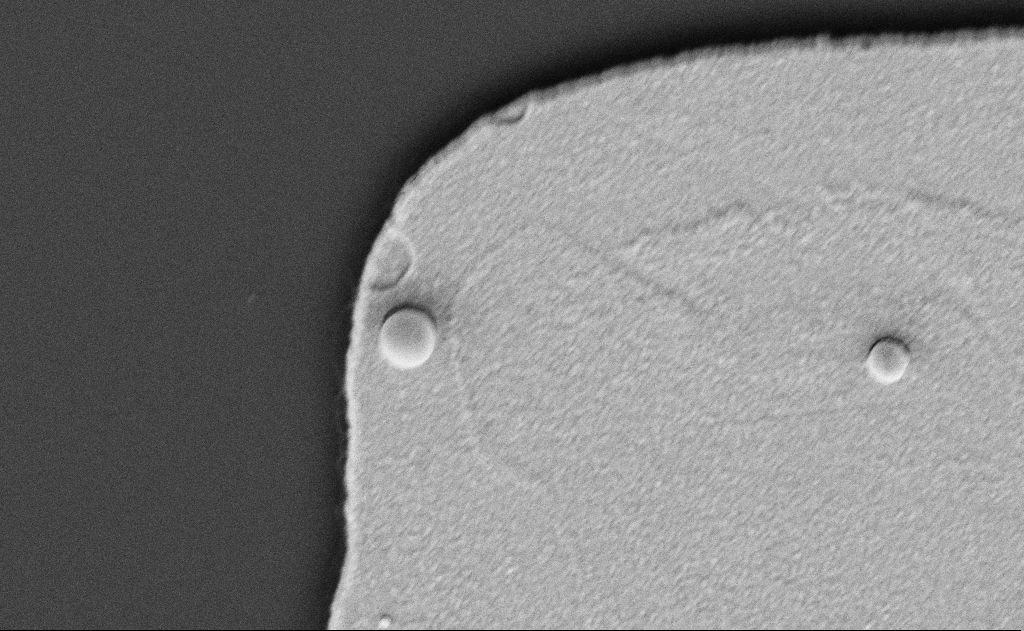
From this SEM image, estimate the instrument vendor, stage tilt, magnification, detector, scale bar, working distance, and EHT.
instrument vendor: Zeiss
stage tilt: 30.1°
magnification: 54.72 K X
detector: SE2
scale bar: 1000 nm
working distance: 6 mm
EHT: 5 kV